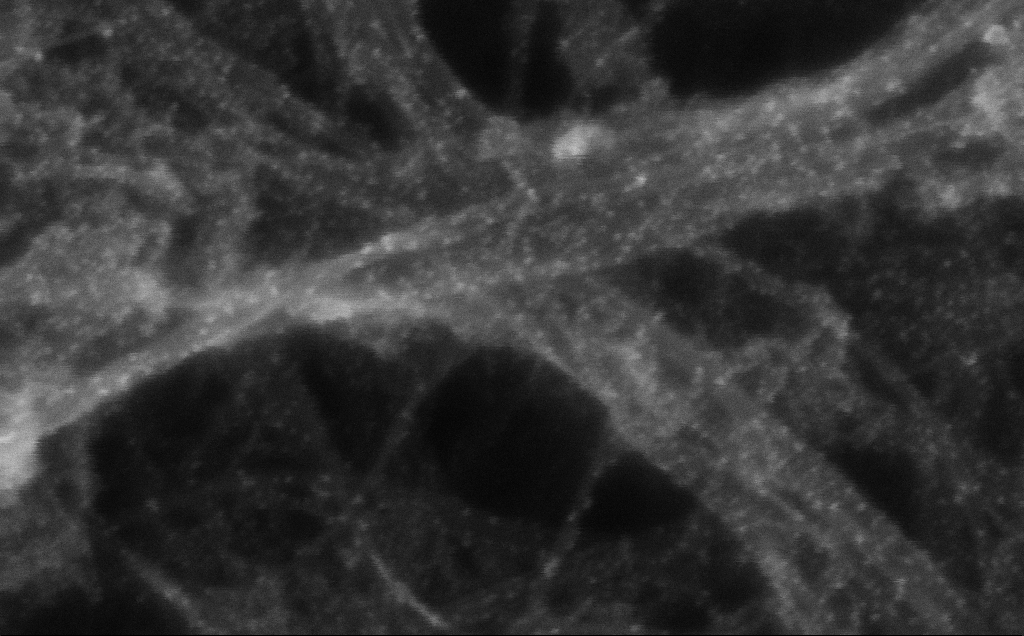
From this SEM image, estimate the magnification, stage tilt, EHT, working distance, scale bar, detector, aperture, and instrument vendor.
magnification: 609.25 K X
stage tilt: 0°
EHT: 10 kV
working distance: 3 mm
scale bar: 100 nm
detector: InLens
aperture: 30 µm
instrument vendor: Zeiss